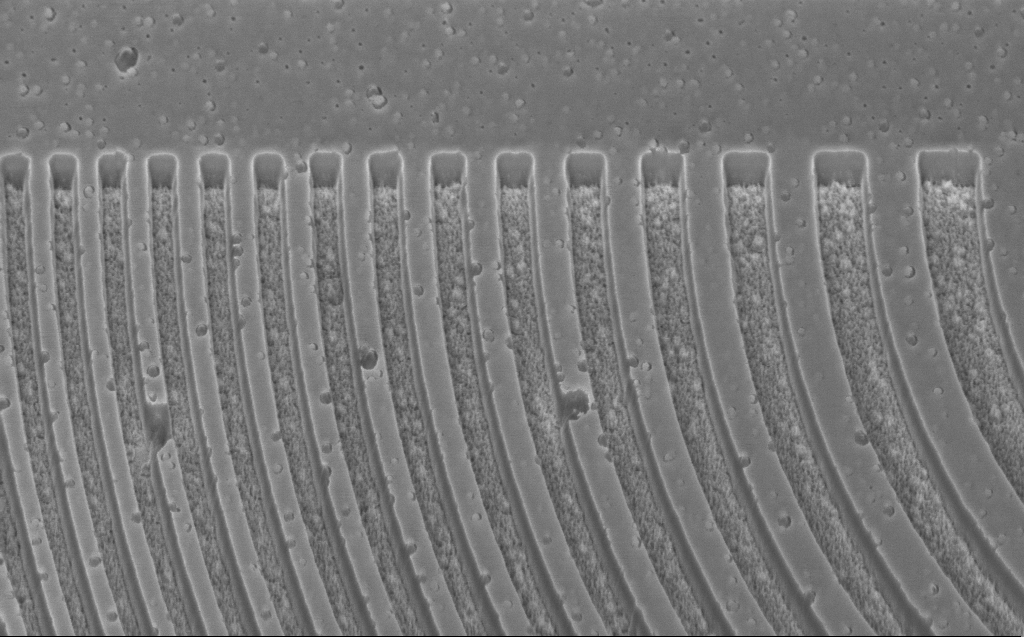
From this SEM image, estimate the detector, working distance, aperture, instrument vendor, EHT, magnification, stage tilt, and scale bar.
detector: InLens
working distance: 6 mm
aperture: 30 µm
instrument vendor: Zeiss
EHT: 3 kV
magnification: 25.44 K X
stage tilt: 45°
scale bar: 2000 nm